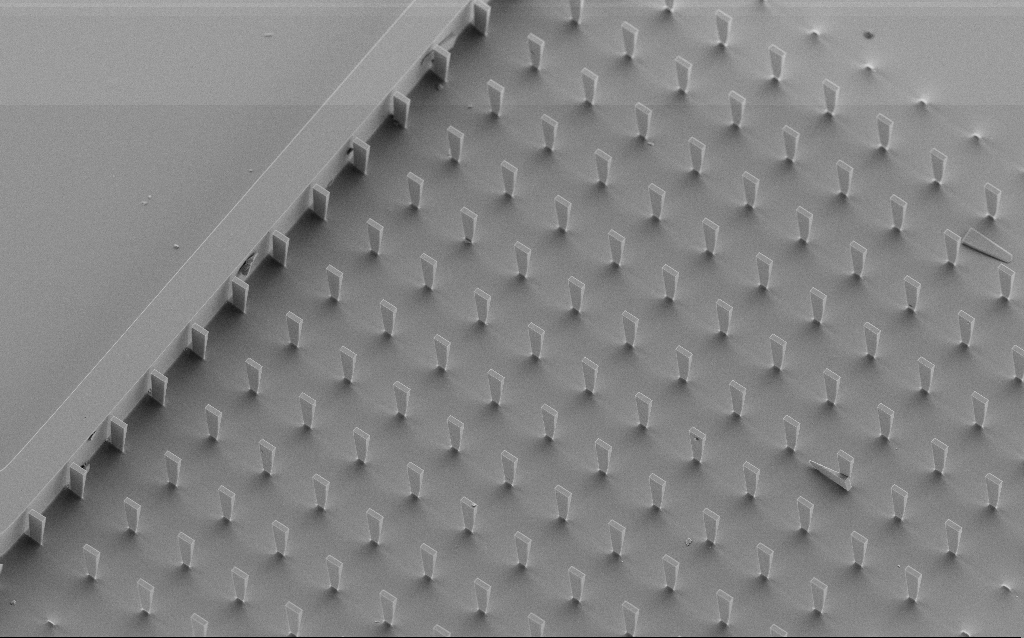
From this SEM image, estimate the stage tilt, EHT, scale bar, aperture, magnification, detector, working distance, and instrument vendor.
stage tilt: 30°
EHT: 5 kV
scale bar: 20000 nm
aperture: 30 µm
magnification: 0.828 K X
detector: SE2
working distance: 6.7 mm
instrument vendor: Zeiss